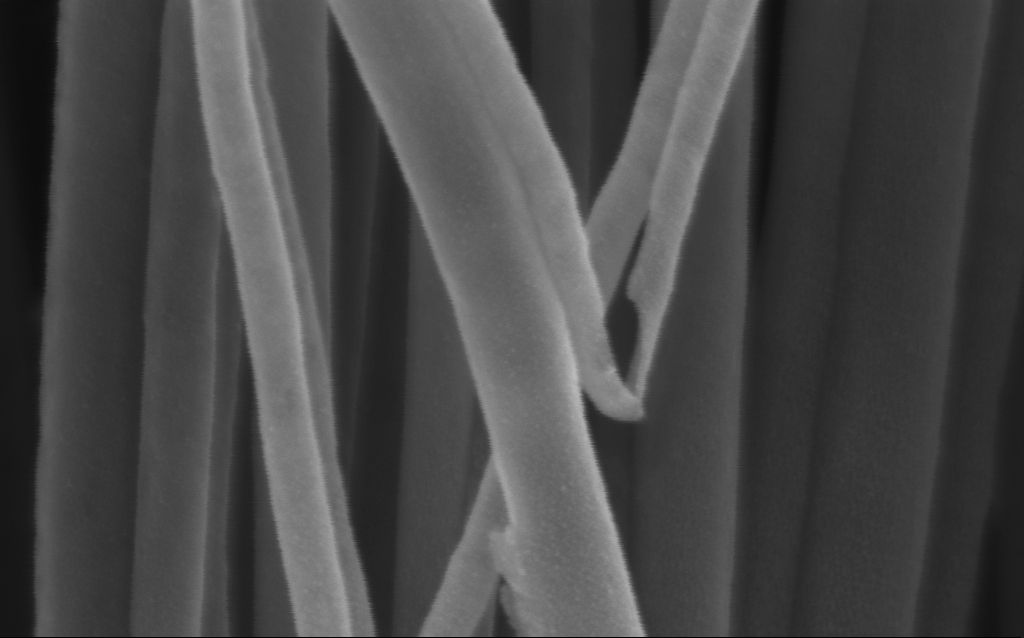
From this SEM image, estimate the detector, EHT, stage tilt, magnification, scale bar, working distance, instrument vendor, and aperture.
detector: InLens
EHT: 3 kV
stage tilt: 0°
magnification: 253.53 K X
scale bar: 200 nm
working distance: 3 mm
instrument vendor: Zeiss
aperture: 30 µm